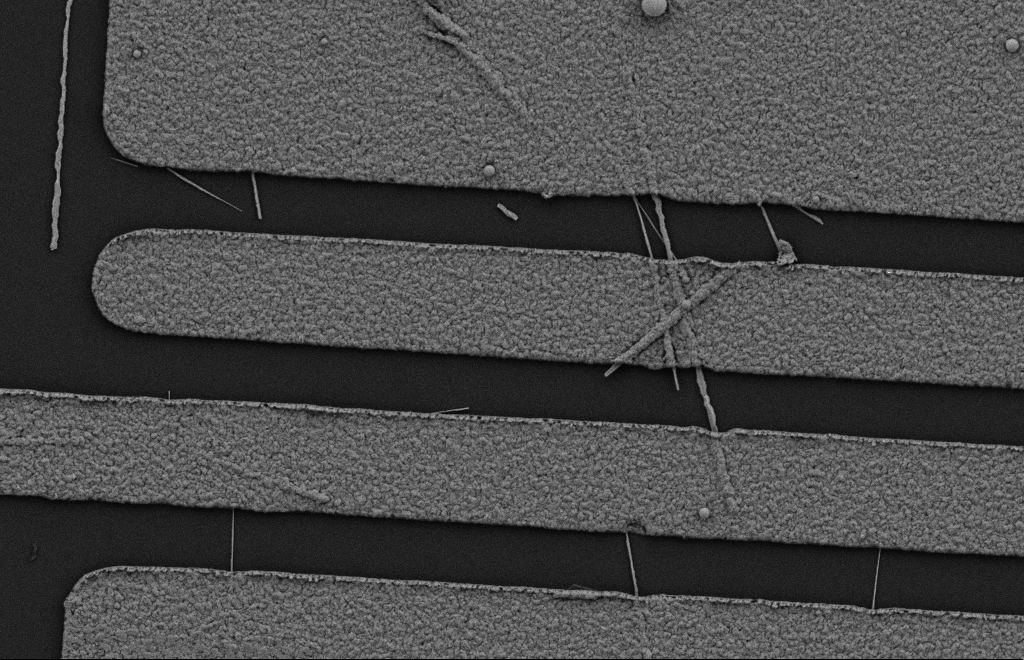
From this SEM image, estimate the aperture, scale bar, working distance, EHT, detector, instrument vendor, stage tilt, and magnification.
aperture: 20 µm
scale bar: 2000 nm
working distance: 10 mm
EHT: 2 kV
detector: SE2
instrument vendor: Zeiss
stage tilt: -0.3°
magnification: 15.42 K X